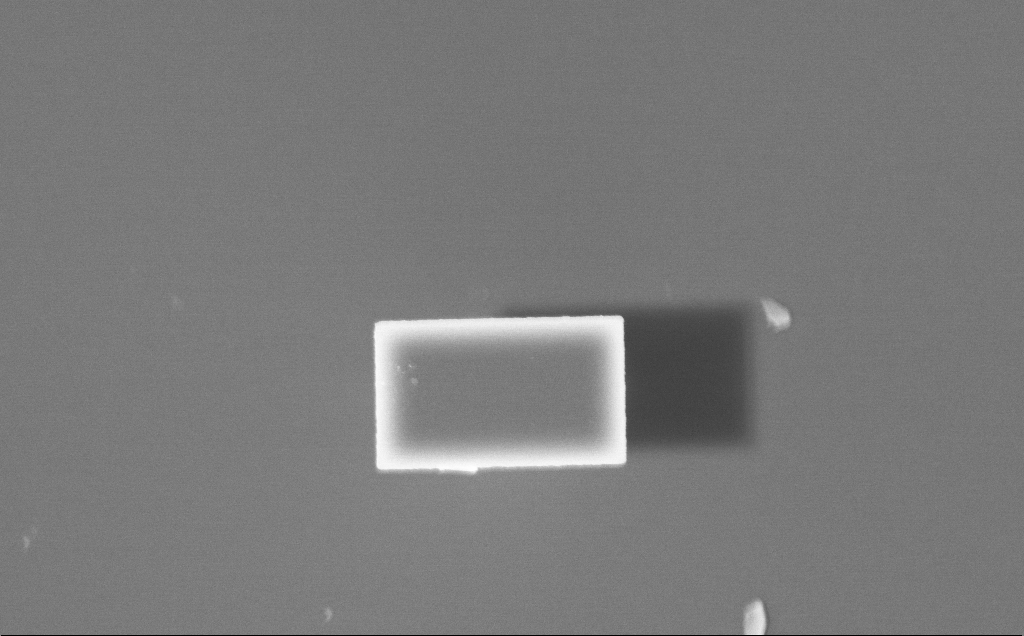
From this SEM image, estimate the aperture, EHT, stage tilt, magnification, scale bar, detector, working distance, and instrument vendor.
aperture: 30 µm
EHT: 7.5 kV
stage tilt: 0°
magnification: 18.91 K X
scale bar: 2000 nm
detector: InLens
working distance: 4 mm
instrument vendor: Zeiss